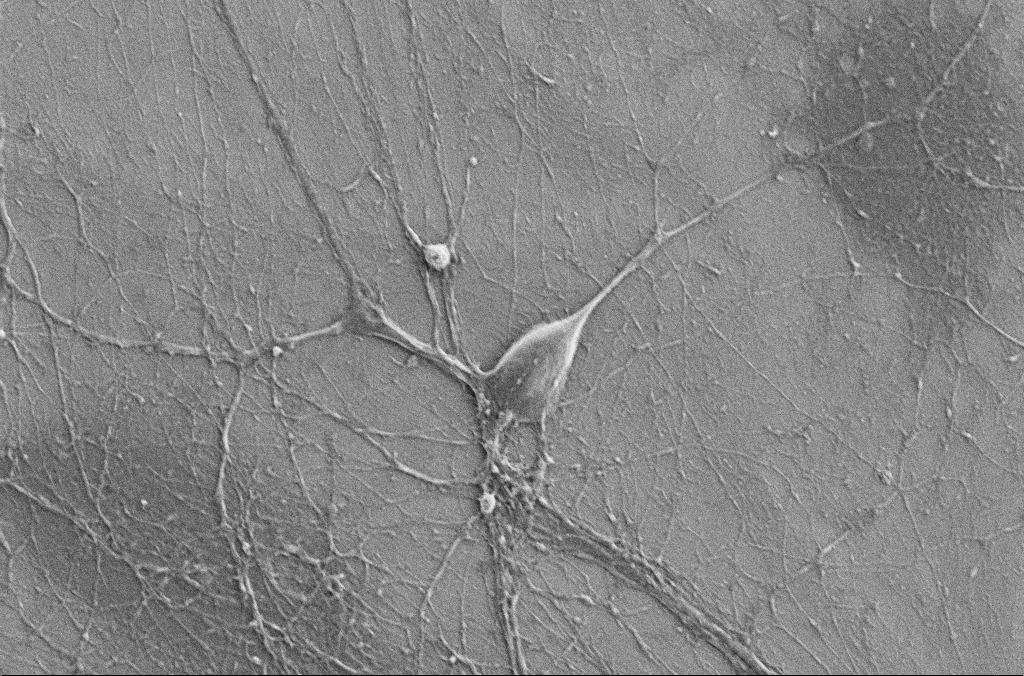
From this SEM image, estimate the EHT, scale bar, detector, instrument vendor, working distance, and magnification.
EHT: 5 kV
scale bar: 20000 nm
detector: SE2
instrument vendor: Zeiss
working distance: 5.9 mm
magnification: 3 K X